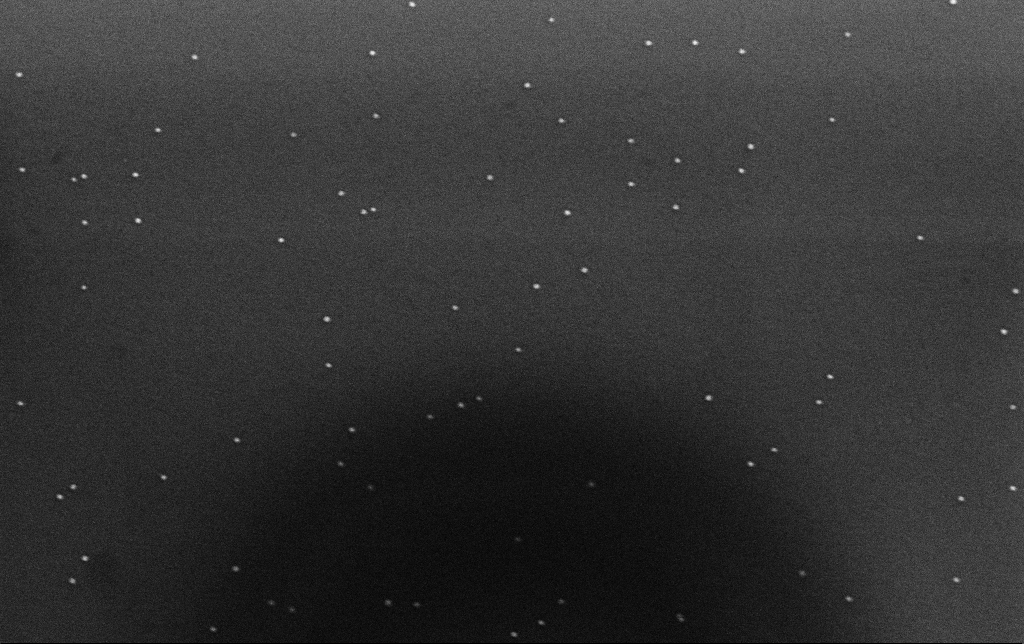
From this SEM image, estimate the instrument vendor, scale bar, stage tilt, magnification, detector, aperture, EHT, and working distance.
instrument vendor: Zeiss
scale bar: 200 nm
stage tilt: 0°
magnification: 100 K X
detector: InLens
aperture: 30 µm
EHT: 10 kV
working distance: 3.1 mm